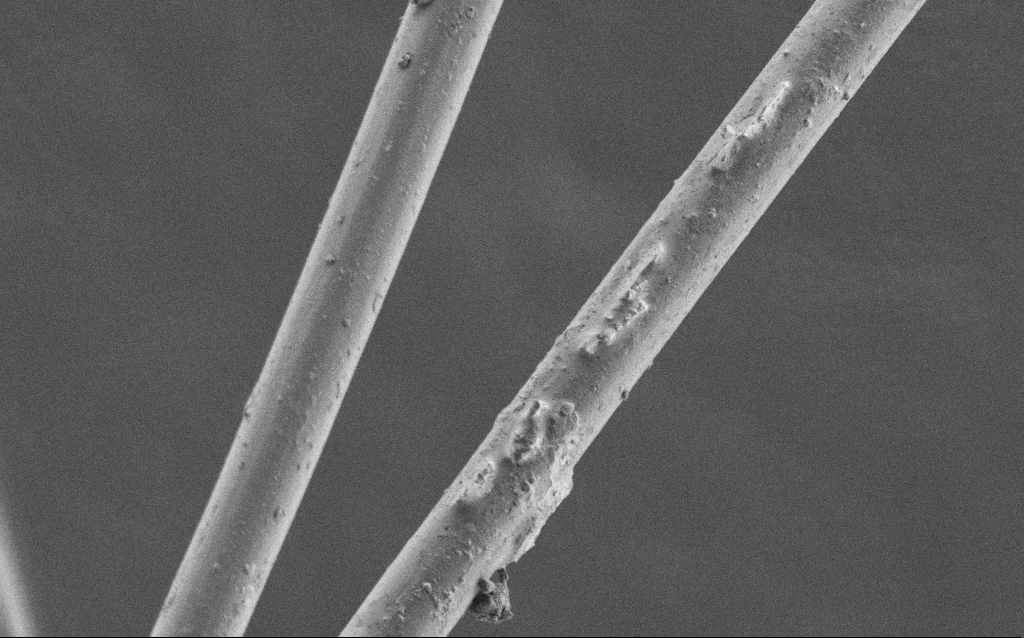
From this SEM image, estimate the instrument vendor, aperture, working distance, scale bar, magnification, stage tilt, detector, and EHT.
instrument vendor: Zeiss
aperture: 30 µm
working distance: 5 mm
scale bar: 20000 nm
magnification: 1.4 K X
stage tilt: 0°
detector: SE2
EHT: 1 kV